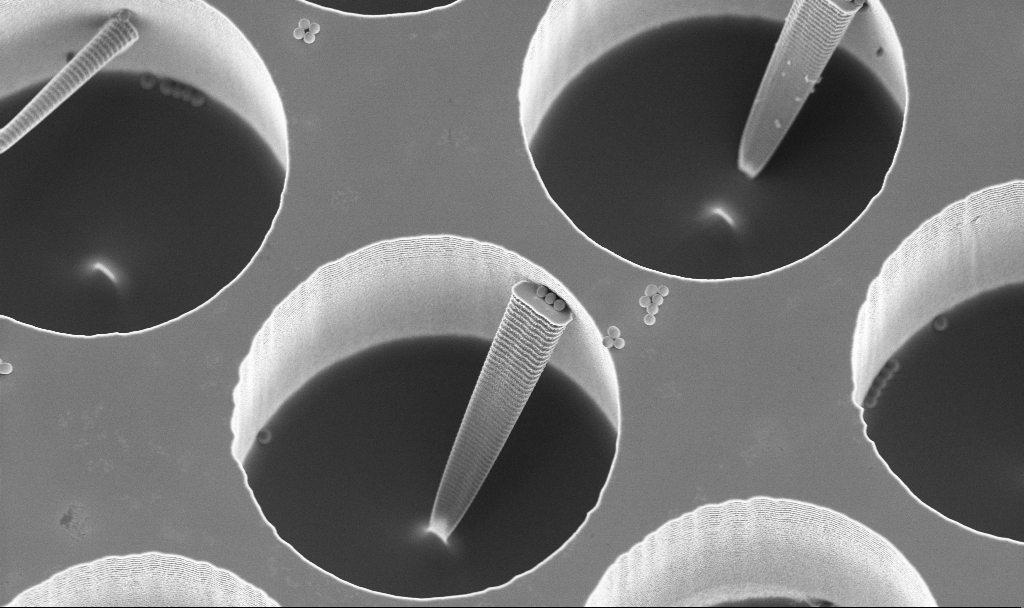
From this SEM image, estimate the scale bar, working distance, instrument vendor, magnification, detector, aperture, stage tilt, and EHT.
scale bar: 10000 nm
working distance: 3 mm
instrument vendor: Zeiss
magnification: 7.05 K X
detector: InLens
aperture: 30 µm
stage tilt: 20°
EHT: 3 kV